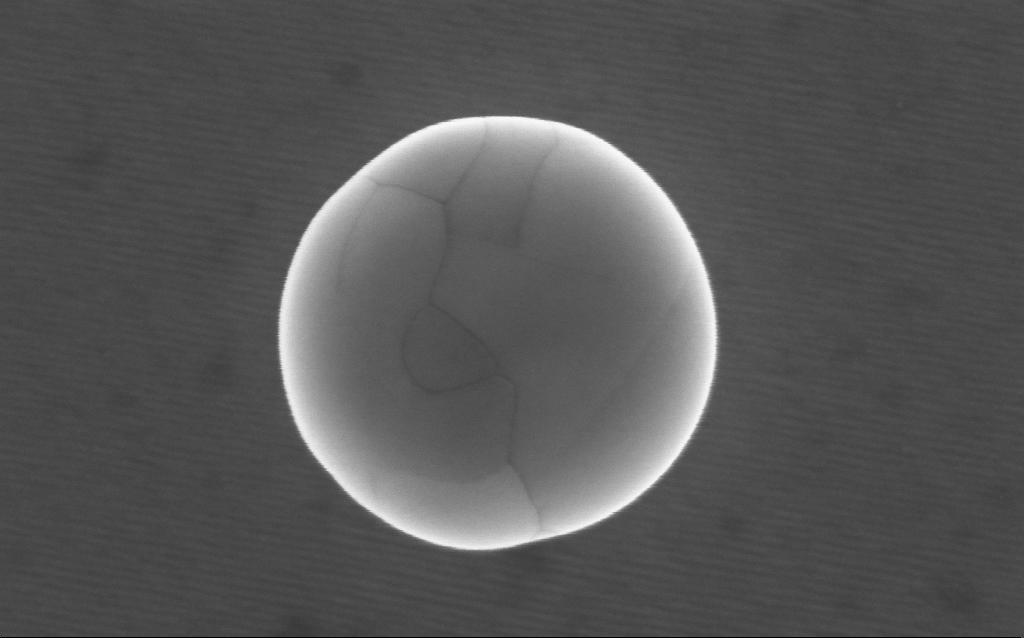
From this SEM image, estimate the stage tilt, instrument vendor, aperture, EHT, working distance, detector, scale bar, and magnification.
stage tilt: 0°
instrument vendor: Zeiss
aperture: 30 µm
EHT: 5 kV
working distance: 3 mm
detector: InLens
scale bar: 100 nm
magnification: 245.72 K X